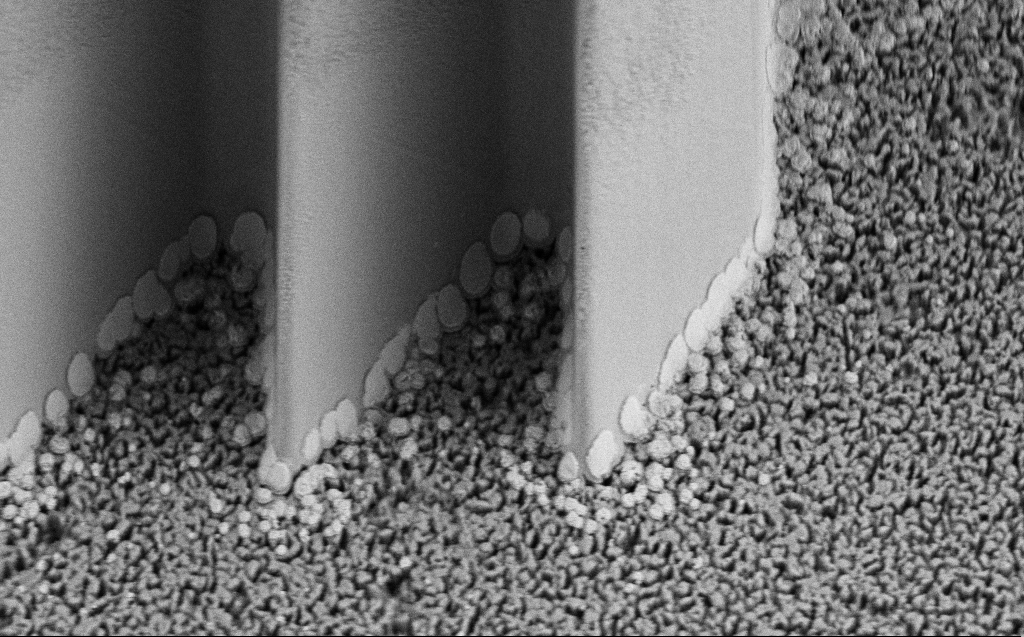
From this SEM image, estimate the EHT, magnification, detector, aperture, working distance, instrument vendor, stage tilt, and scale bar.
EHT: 5 kV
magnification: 9.57 K X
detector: SE2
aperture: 30 µm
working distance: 5 mm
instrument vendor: Zeiss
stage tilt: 45°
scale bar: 2000 nm